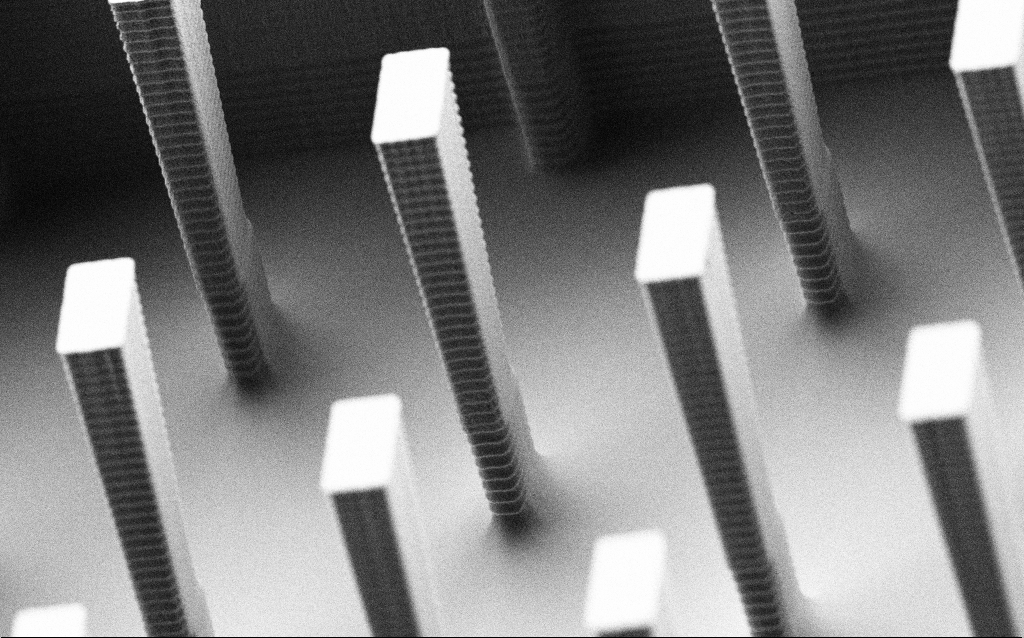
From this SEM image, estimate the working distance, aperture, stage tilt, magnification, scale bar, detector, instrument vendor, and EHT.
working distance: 3.2 mm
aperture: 30 µm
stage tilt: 50°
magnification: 12.41 K X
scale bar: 1000 nm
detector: SE2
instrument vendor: Zeiss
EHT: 1.5 kV